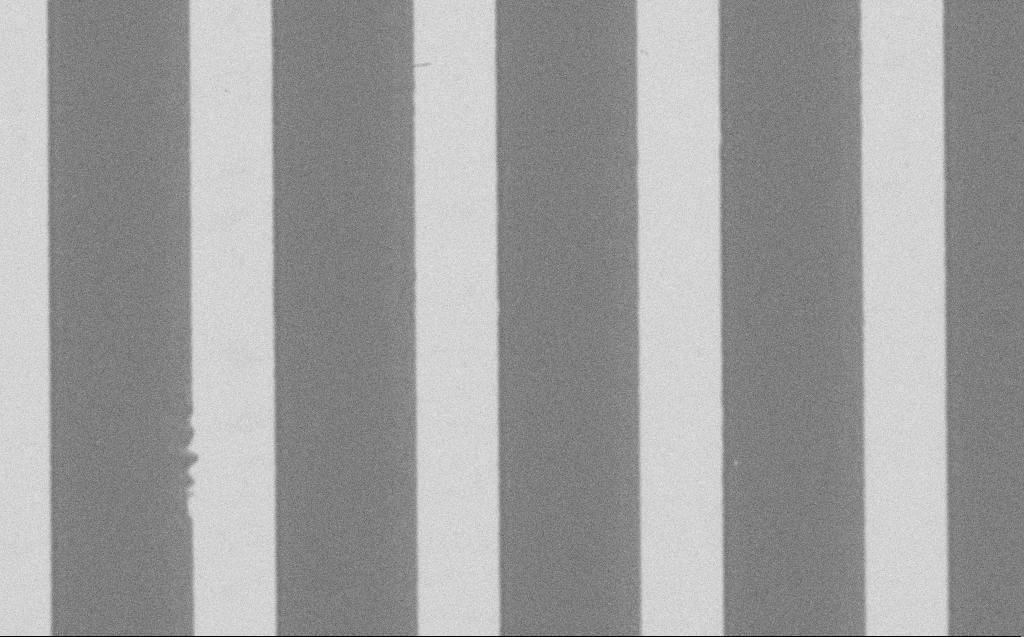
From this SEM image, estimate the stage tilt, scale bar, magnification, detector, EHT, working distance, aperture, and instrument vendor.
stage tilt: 0°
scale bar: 20000 nm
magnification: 2.97 K X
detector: SE2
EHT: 1.2 kV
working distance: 6 mm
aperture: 30 µm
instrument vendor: Zeiss